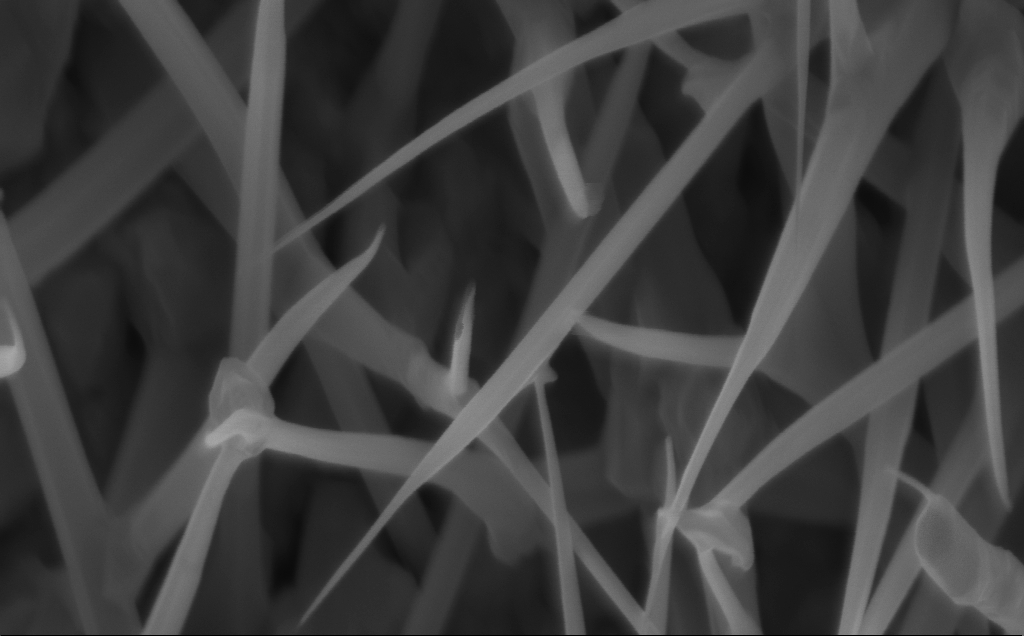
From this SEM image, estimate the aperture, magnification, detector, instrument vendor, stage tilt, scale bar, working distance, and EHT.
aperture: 30 µm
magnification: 128.62 K X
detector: InLens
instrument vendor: Zeiss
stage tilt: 0°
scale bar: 200 nm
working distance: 5 mm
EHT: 5 kV